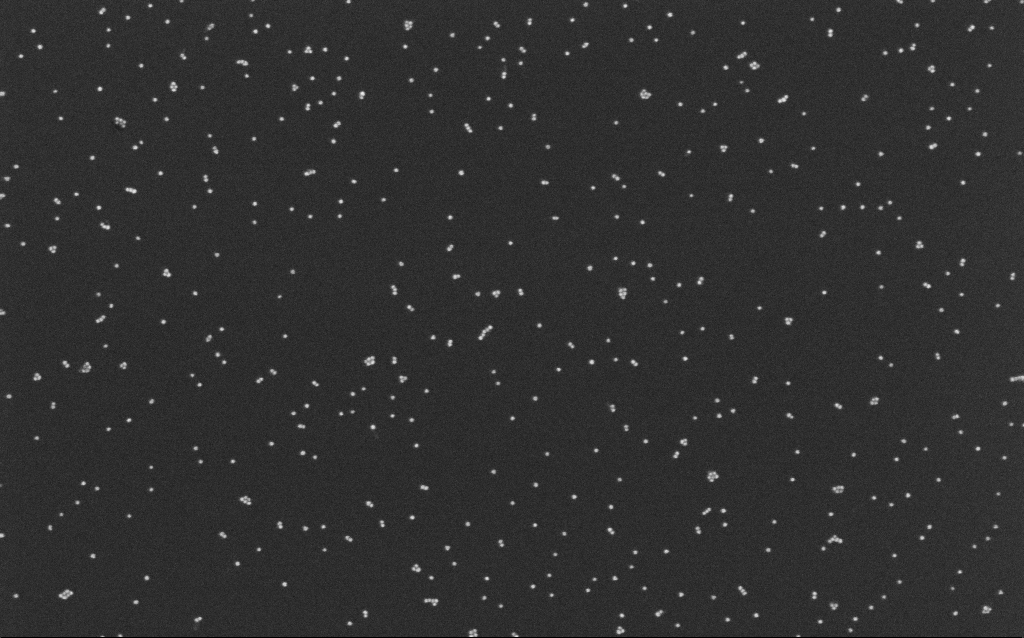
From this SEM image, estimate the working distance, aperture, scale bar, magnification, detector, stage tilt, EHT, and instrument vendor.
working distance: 6.5 mm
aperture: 30 µm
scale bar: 200 nm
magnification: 100 K X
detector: InLens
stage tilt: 0°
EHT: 10 kV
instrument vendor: Zeiss